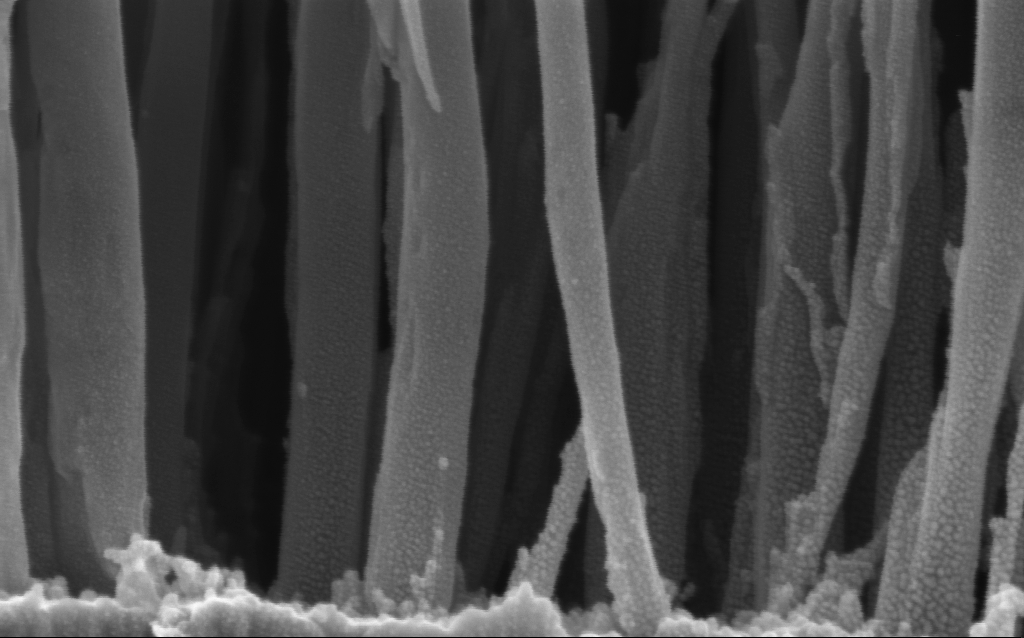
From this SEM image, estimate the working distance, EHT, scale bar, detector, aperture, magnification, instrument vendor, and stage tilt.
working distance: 3 mm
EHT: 3 kV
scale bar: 100 nm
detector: InLens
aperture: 30 µm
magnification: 196.18 K X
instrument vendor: Zeiss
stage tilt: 0°